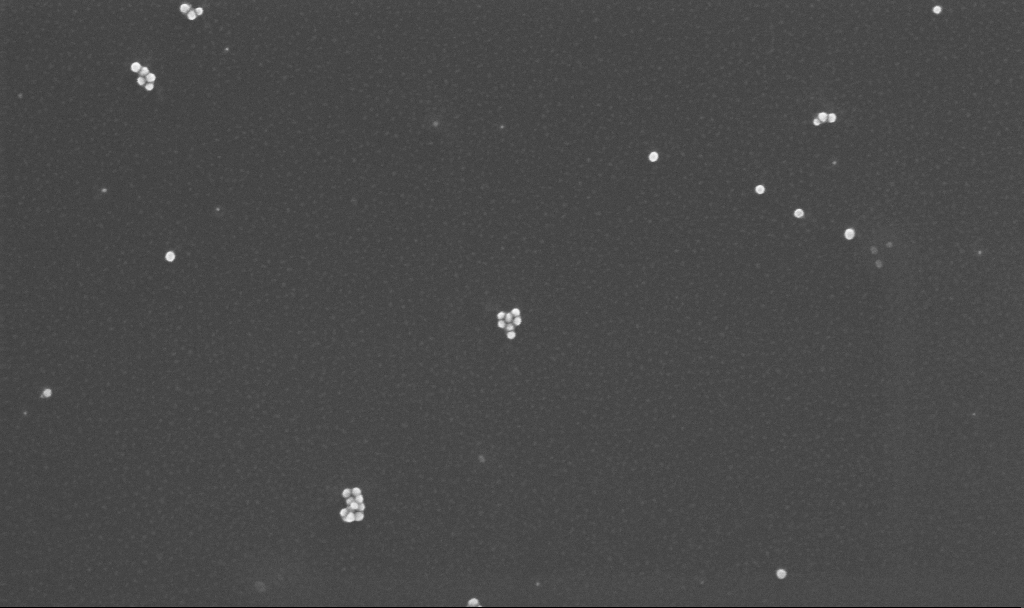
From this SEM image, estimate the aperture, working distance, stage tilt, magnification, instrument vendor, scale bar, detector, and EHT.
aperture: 30 µm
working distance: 3.2 mm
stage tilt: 0°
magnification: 150 K X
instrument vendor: Zeiss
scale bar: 100 nm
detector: InLens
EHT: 10 kV